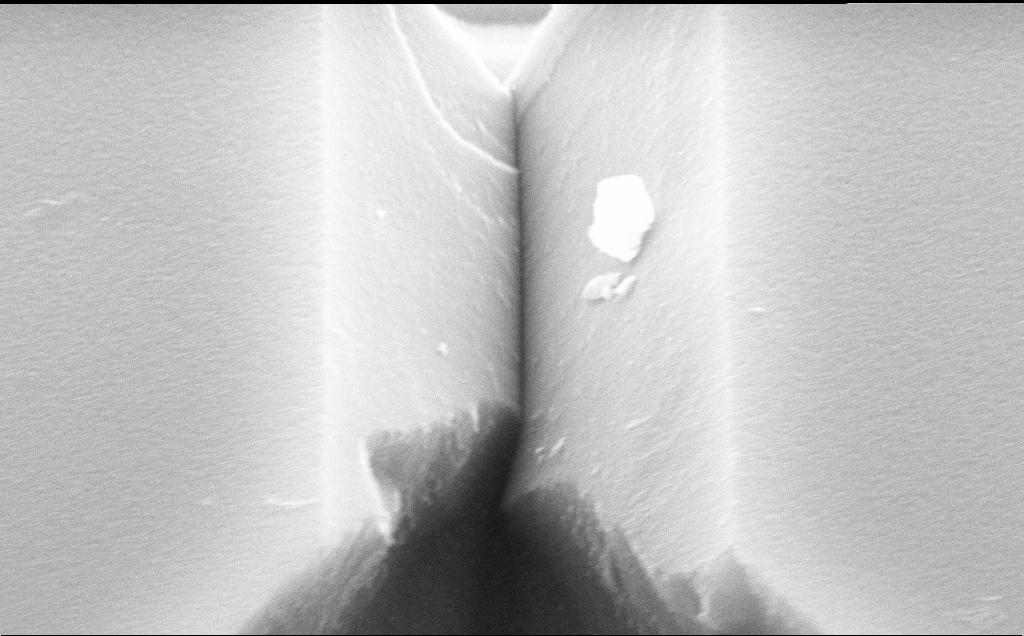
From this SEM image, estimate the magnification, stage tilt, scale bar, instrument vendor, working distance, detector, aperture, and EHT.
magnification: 66.07 K X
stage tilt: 50°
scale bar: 1000 nm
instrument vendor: Zeiss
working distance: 10 mm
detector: SE2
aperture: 30 µm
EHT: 10 kV